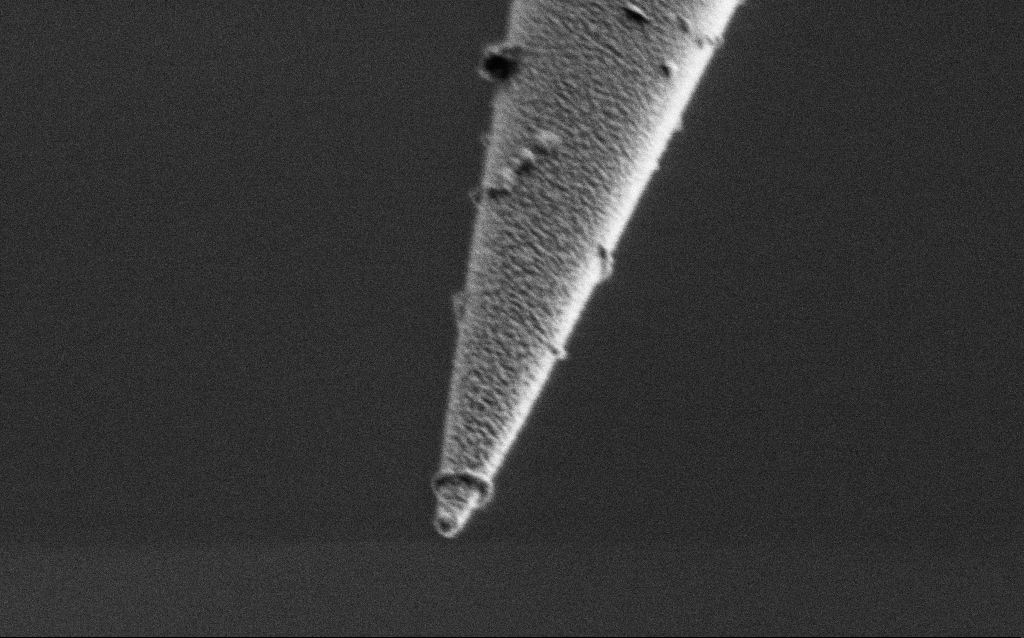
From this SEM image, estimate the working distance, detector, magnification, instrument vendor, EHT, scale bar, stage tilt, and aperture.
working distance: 6.5 mm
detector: SE2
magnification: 75 K X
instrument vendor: Zeiss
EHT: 1 kV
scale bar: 200 nm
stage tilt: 45°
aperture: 30 µm